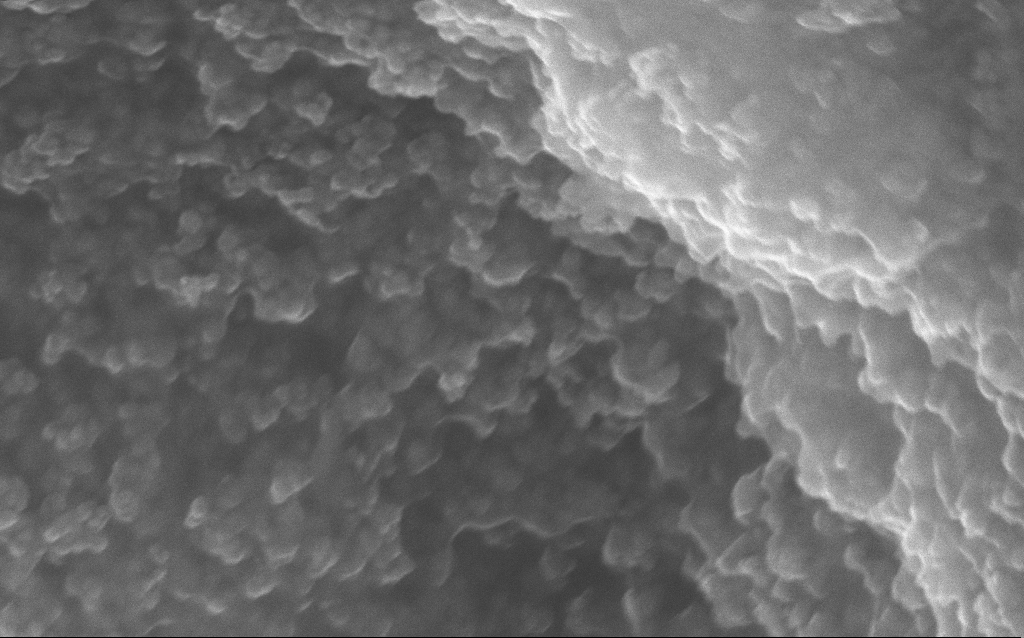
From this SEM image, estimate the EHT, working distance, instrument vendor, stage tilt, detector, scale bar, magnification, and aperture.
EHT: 10 kV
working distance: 2.8 mm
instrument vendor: Zeiss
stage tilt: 0°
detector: InLens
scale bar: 100 nm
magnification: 211.33 K X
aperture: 30 µm